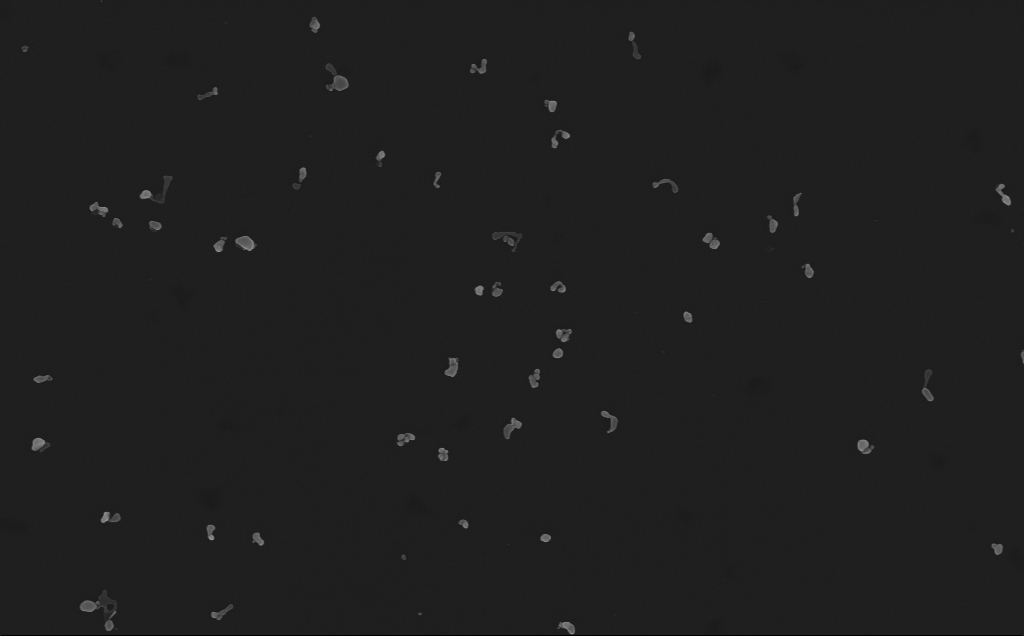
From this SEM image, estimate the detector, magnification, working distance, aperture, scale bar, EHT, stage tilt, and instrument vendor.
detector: InLens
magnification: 48.86 K X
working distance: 3 mm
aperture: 30 µm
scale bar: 1000 nm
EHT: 10 kV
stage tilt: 0°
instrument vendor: Zeiss